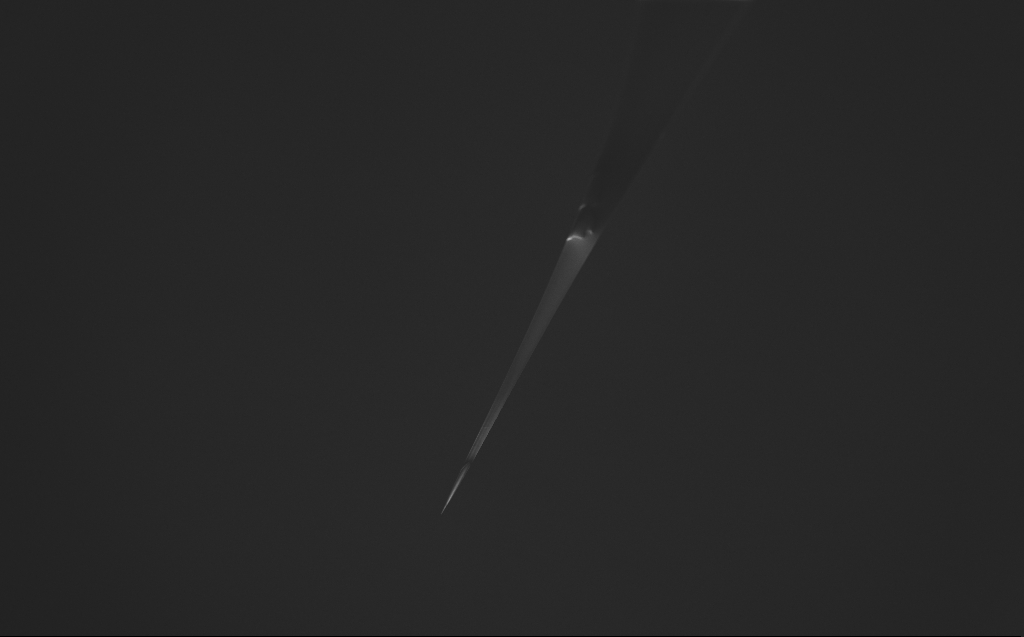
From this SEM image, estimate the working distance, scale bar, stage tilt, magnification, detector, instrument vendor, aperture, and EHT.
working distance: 6 mm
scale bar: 200000 nm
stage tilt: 45°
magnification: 0.099 K X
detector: InLens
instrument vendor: Zeiss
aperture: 30 µm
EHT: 2 kV